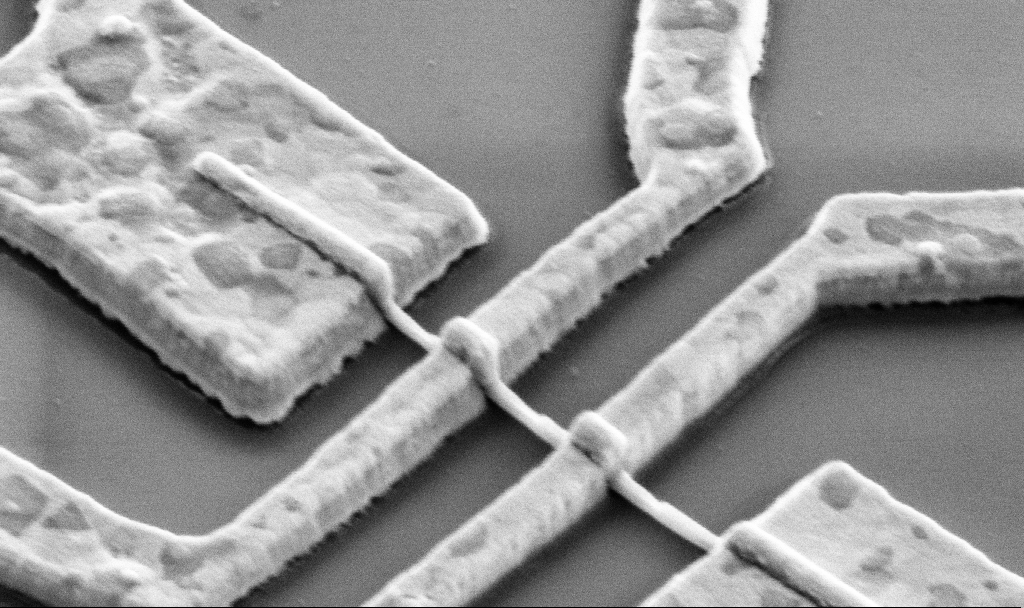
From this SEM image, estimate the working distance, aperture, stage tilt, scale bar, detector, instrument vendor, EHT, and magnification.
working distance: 14.2 mm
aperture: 30 µm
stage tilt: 45°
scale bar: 1000 nm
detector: SE2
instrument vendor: Zeiss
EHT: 5 kV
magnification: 60 K X